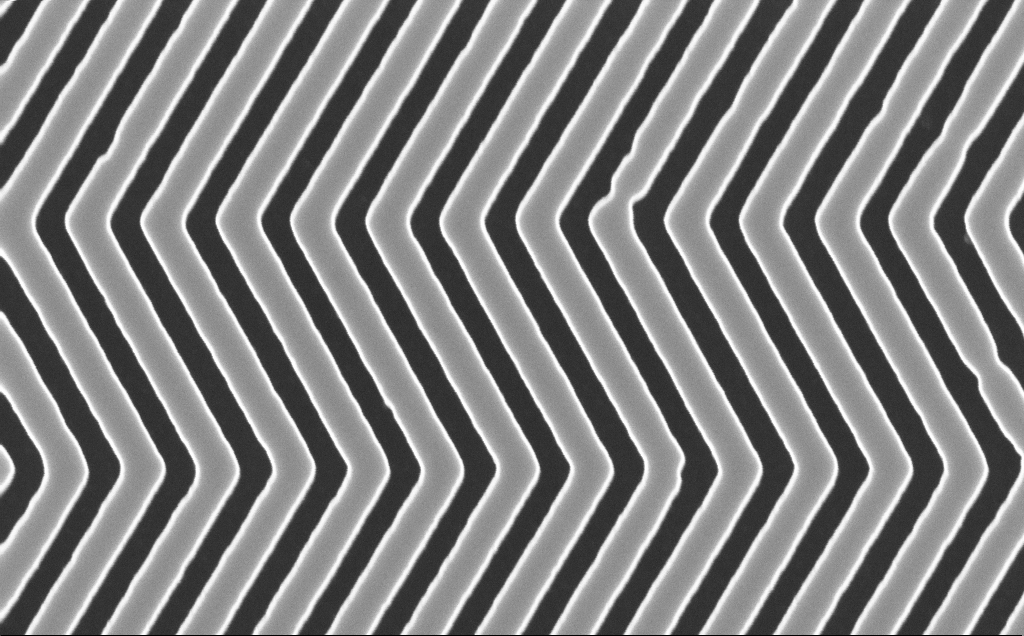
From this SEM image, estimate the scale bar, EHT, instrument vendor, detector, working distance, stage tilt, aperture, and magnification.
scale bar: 200 nm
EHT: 10 kV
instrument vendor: Zeiss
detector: InLens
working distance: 6 mm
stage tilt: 0°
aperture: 30 µm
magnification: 91.67 K X